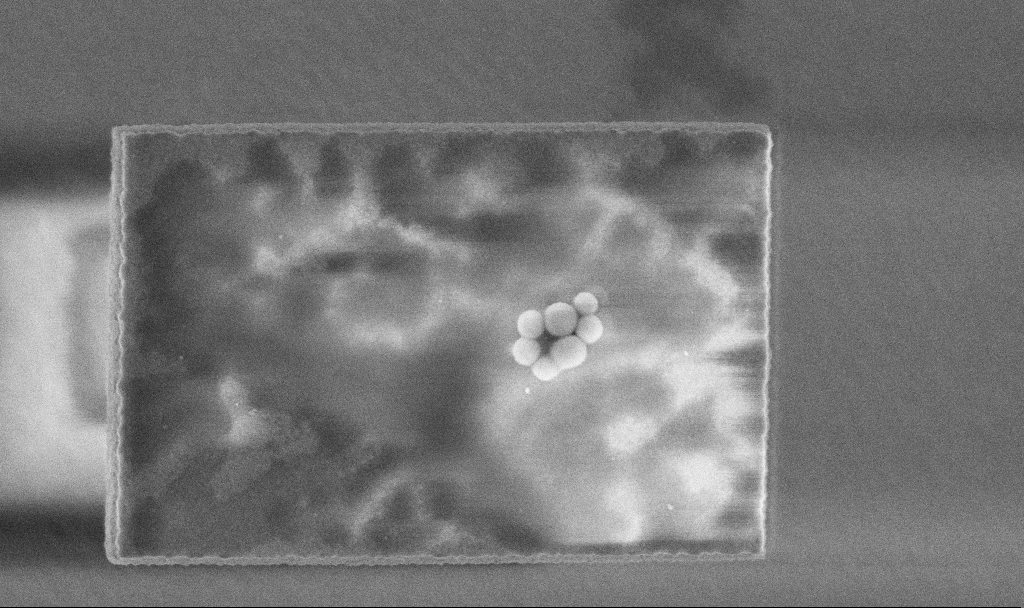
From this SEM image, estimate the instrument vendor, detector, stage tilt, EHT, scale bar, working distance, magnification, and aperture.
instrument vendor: Zeiss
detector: InLens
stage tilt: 0°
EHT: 3 kV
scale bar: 1000 nm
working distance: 3.3 mm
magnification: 53.19 K X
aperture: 30 µm